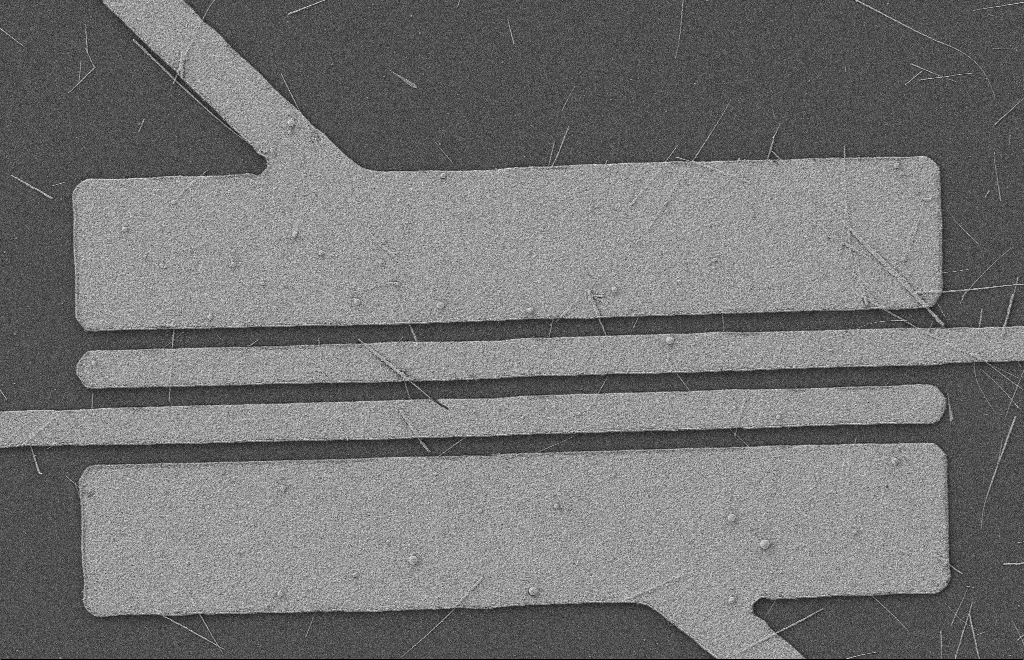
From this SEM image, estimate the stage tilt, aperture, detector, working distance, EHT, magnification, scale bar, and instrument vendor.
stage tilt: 0°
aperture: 20 µm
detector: SE2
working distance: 8 mm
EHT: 2 kV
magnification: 5.24 K X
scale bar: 2000 nm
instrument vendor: Zeiss